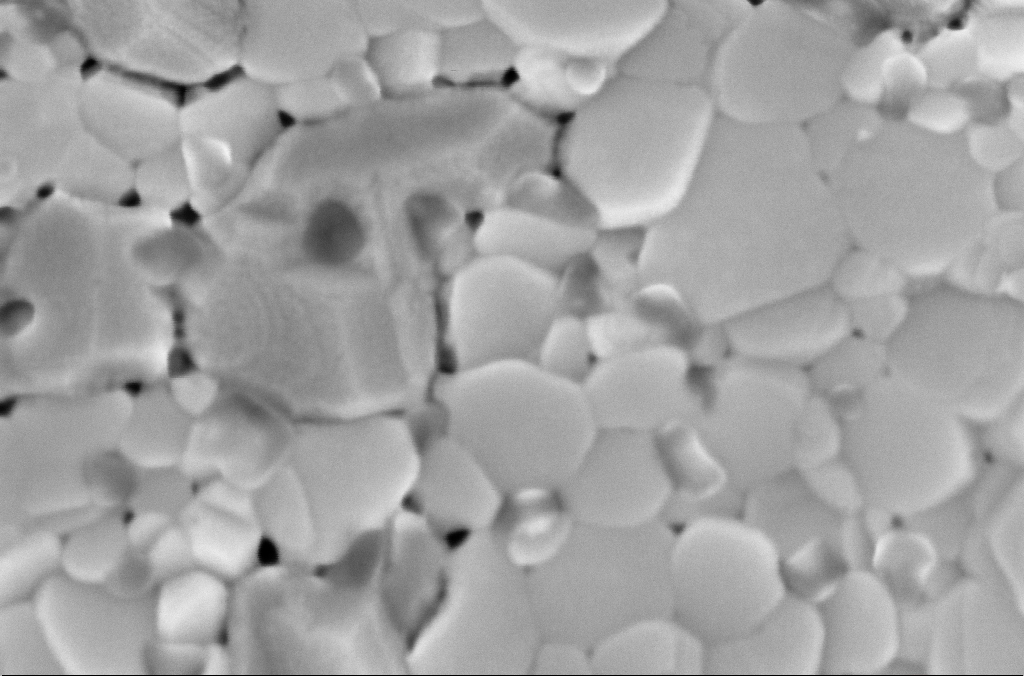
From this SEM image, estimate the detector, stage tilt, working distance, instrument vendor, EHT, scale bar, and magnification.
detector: InLens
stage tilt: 0°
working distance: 3 mm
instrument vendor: Zeiss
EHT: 5 kV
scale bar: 200 nm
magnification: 208.49 K X